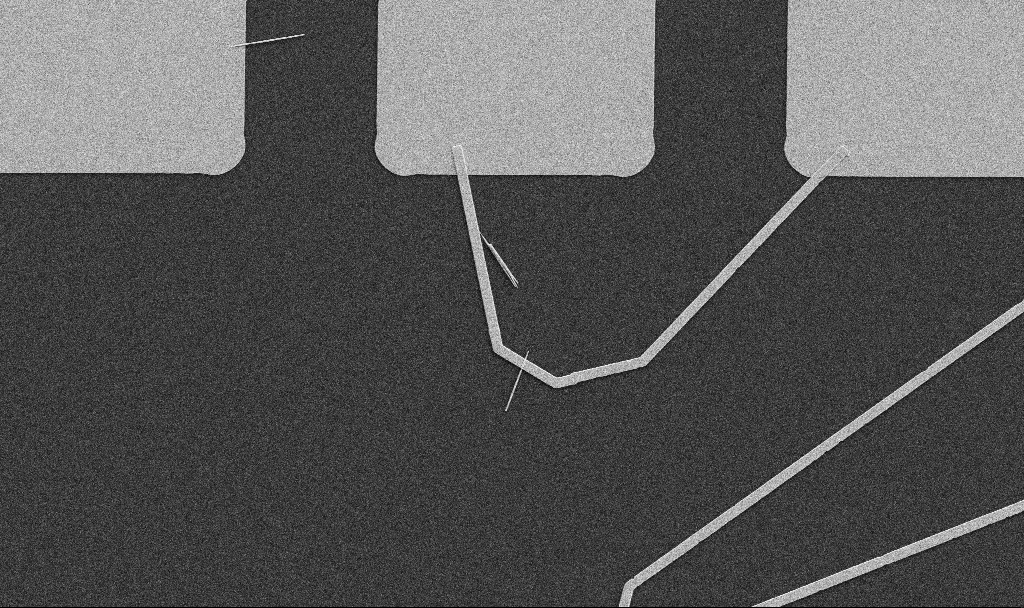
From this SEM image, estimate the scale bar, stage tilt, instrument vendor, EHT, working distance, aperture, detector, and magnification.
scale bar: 10000 nm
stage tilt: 0°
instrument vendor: Zeiss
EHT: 5 kV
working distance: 10.7 mm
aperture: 30 µm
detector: SE2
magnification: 5 K X